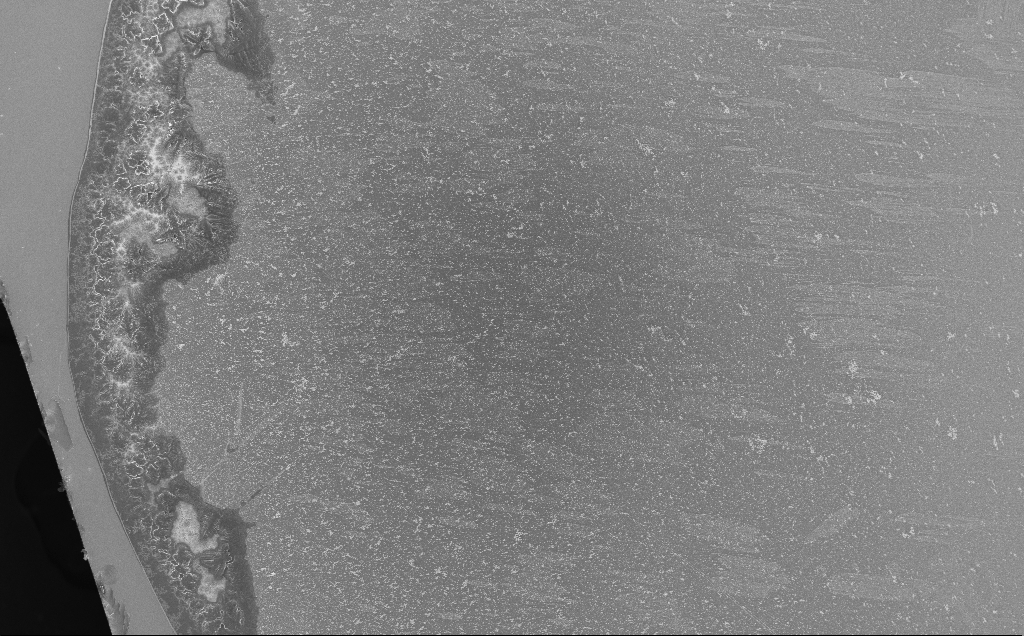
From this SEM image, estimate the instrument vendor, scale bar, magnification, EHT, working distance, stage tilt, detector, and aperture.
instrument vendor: Zeiss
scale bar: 100000 nm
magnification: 0.316 K X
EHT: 3 kV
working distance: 5 mm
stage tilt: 0°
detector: InLens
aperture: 30 µm